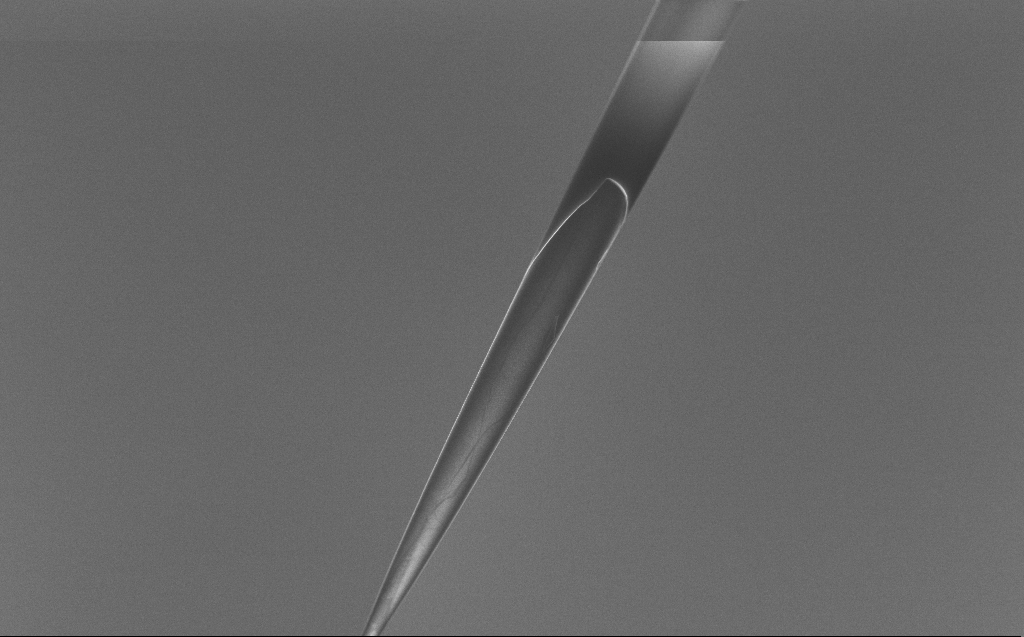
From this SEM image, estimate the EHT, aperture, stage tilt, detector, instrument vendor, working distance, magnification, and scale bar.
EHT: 5 kV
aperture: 30 µm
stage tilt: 45°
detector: InLens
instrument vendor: Zeiss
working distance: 6 mm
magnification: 1 K X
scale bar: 20000 nm